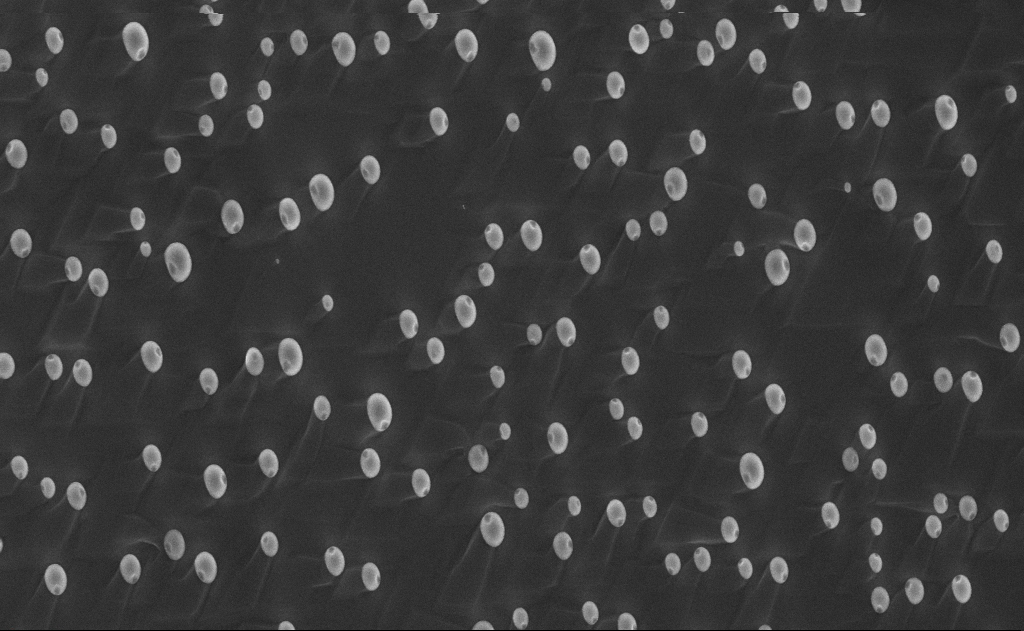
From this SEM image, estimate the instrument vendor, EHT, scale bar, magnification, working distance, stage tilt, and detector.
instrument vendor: Zeiss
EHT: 10 kV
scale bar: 2000 nm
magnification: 10 K X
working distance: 14 mm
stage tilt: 0°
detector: InLens